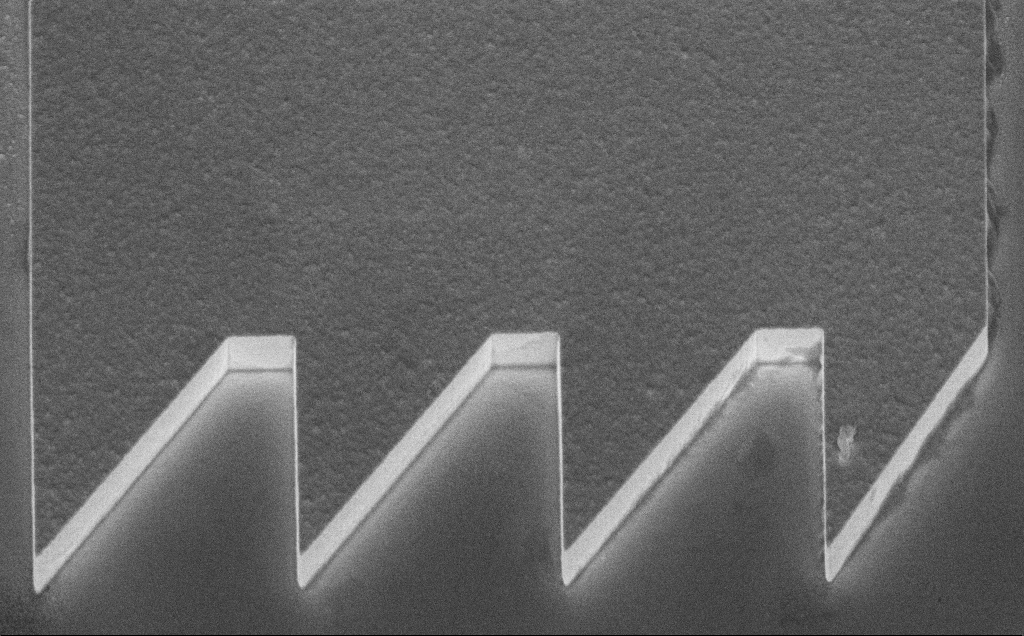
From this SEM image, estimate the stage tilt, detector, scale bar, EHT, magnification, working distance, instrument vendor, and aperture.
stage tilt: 45°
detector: InLens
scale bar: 2000 nm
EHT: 10 kV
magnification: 8.4 K X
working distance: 8 mm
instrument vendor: Zeiss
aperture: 30 µm